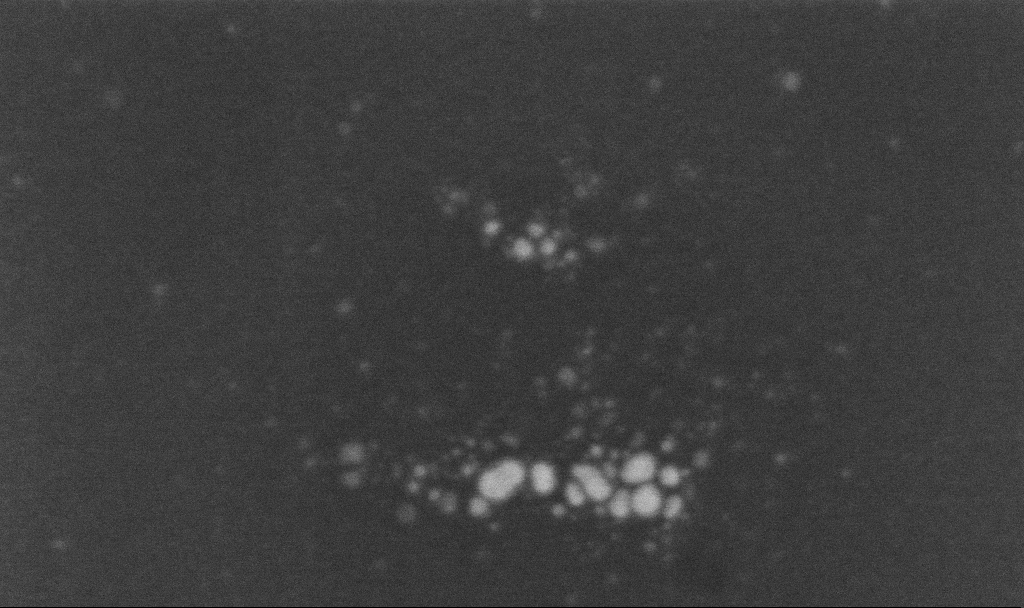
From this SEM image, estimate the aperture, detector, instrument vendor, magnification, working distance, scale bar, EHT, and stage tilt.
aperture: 30 µm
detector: InLens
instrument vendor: Zeiss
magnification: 500 K X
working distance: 3.2 mm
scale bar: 100 nm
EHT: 5 kV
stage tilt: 0°